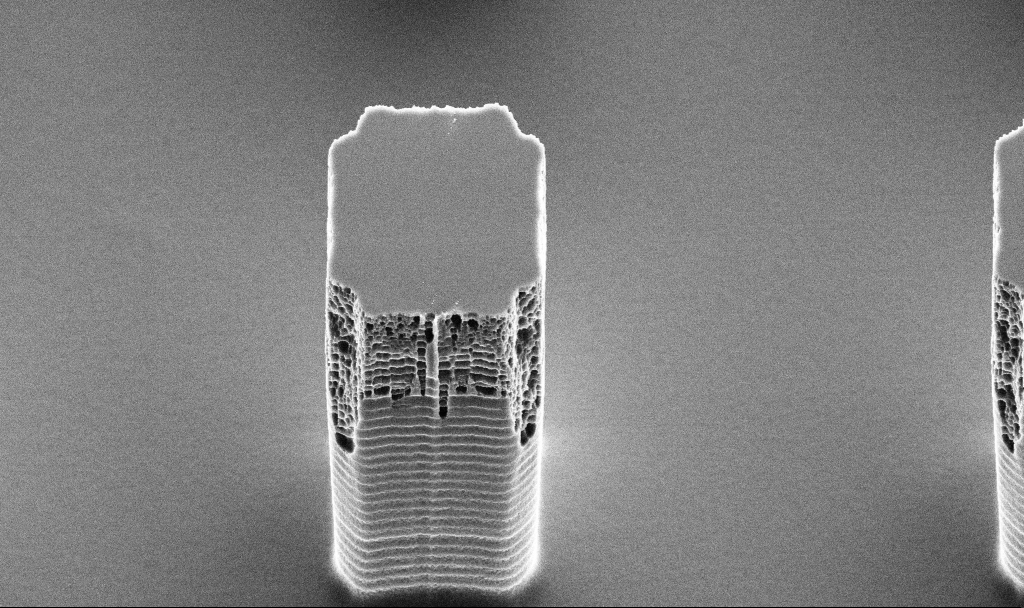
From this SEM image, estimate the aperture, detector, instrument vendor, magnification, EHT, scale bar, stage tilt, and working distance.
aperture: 30 µm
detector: SE2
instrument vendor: Zeiss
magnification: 8.13 K X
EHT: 5 kV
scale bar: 2000 nm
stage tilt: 45°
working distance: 11.2 mm